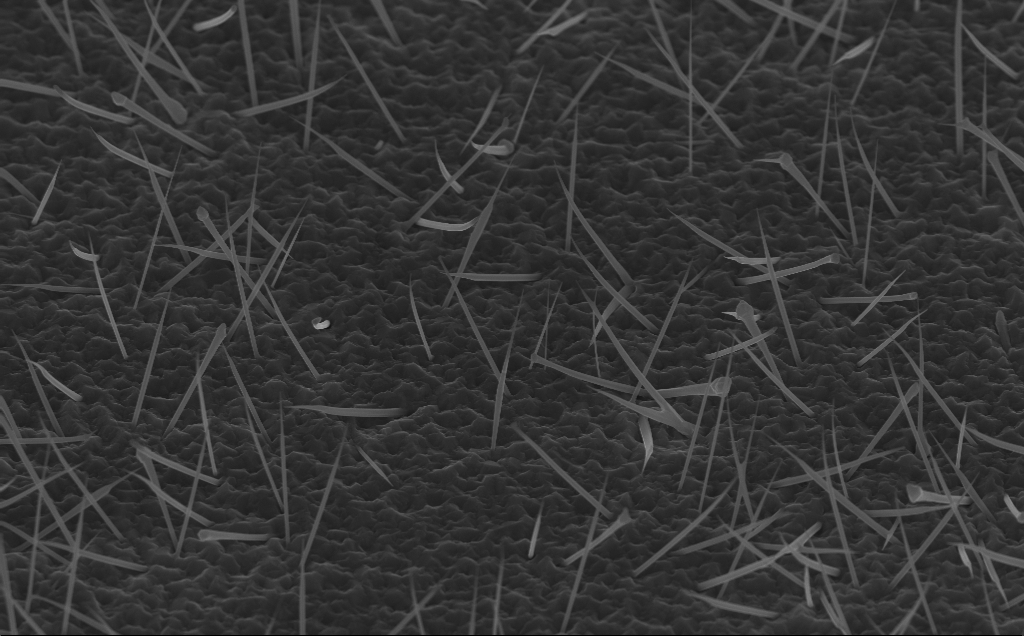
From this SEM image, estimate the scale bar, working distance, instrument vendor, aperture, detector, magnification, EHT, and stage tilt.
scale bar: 2000 nm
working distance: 5 mm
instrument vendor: Zeiss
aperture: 30 µm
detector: InLens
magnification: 20 K X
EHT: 10 kV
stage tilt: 45°